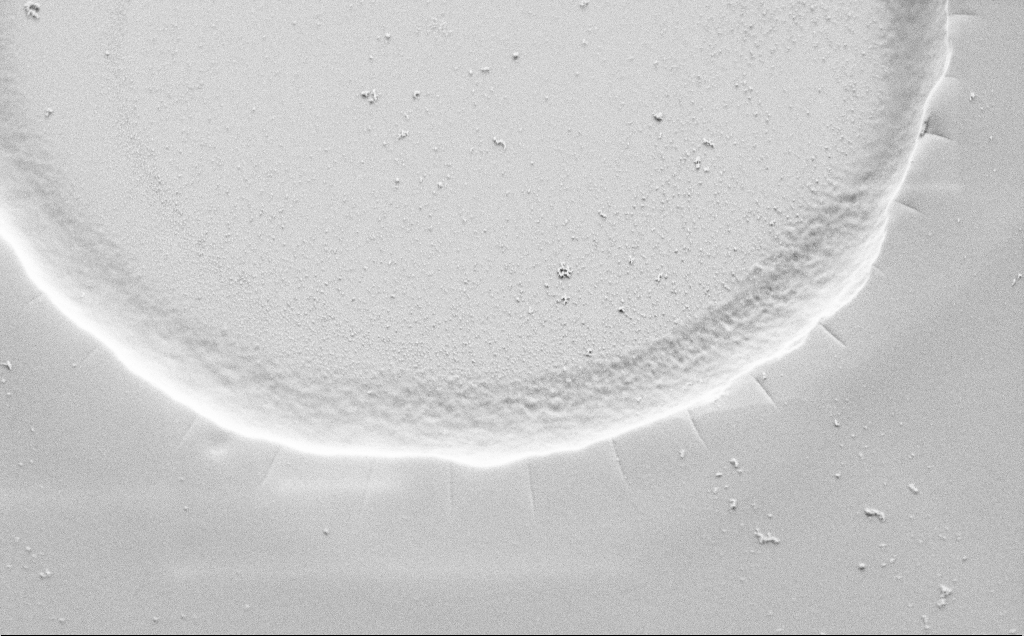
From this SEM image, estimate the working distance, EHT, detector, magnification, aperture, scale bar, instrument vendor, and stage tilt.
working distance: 6 mm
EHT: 1.5 kV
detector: SE2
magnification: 14.23 K X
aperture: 30 µm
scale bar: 2000 nm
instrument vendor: Zeiss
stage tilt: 45°